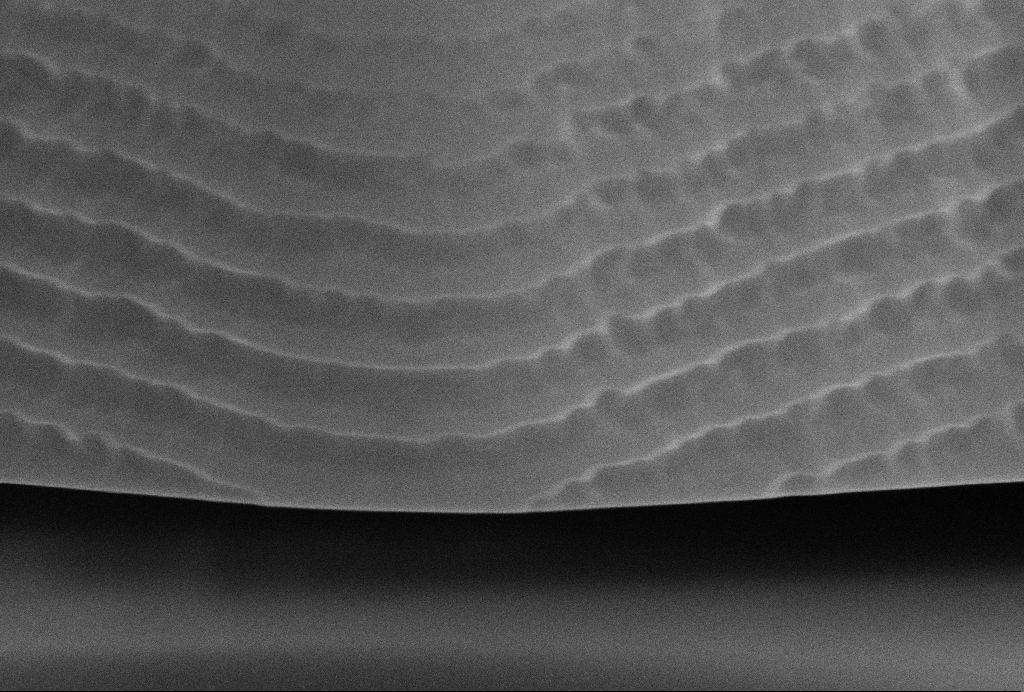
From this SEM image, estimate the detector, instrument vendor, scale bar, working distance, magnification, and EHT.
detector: SE2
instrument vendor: Zeiss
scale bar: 200 nm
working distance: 14 mm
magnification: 88.44 K X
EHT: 10 kV